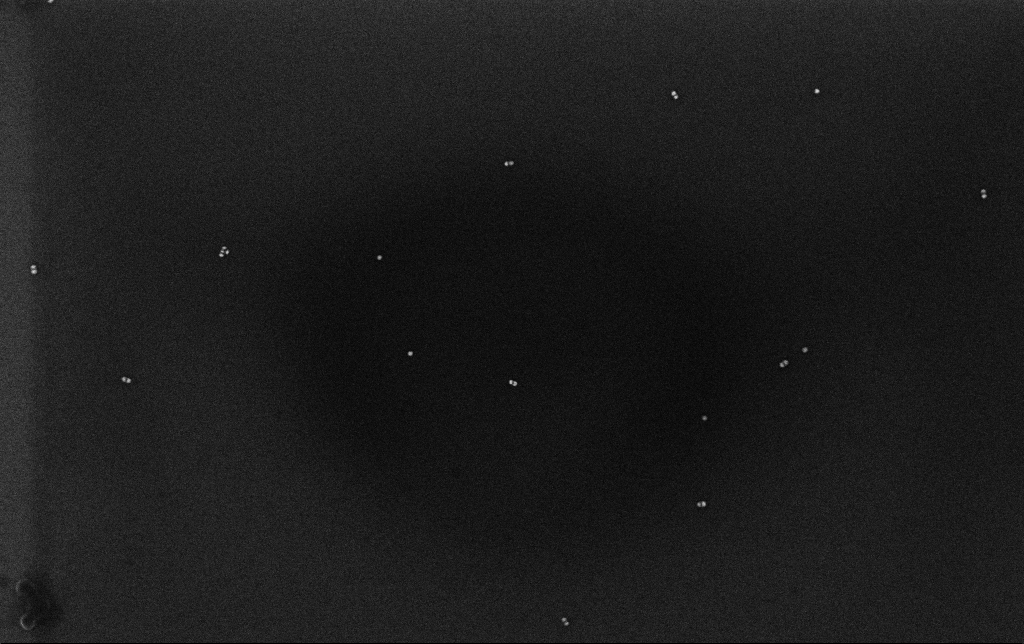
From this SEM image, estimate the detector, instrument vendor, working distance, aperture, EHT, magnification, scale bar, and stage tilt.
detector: InLens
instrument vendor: Zeiss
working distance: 3.2 mm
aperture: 30 µm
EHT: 10 kV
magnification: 100 K X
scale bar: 200 nm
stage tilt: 0°